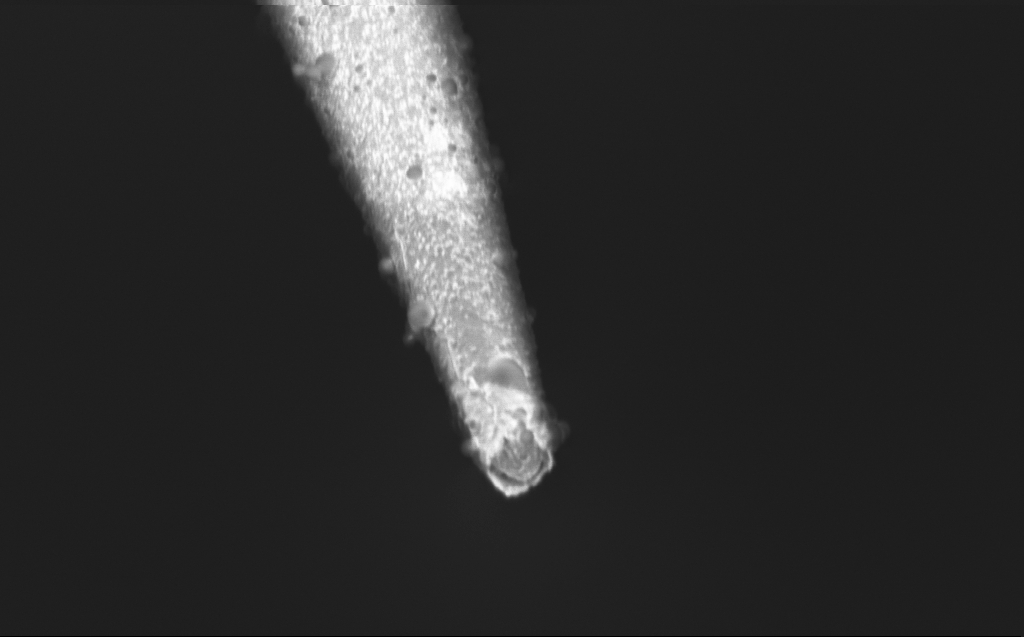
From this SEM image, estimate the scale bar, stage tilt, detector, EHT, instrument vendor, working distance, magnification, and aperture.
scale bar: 1000 nm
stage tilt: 45°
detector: InLens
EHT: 0.8 kV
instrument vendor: Zeiss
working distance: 4 mm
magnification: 50 K X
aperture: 30 µm